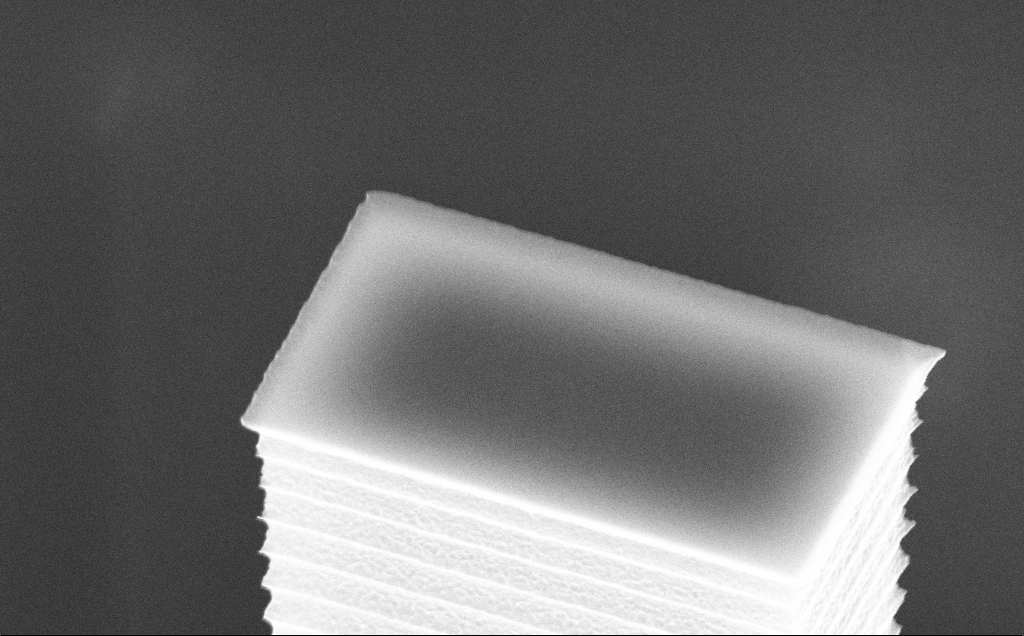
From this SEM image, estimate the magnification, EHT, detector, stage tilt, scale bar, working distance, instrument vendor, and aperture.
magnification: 46.47 K X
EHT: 10 kV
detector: InLens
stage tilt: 45°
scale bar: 1000 nm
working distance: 7 mm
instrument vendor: Zeiss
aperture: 30 µm